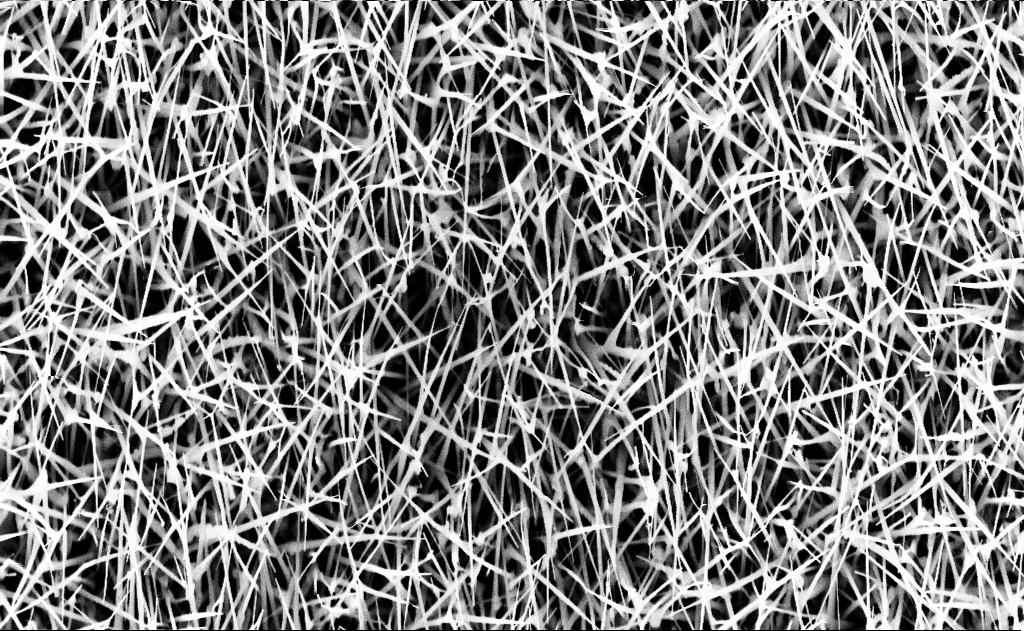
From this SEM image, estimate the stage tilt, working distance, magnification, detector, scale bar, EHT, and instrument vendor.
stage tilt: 0°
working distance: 16 mm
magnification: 20 K X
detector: InLens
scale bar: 1000 nm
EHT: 10 kV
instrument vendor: Zeiss